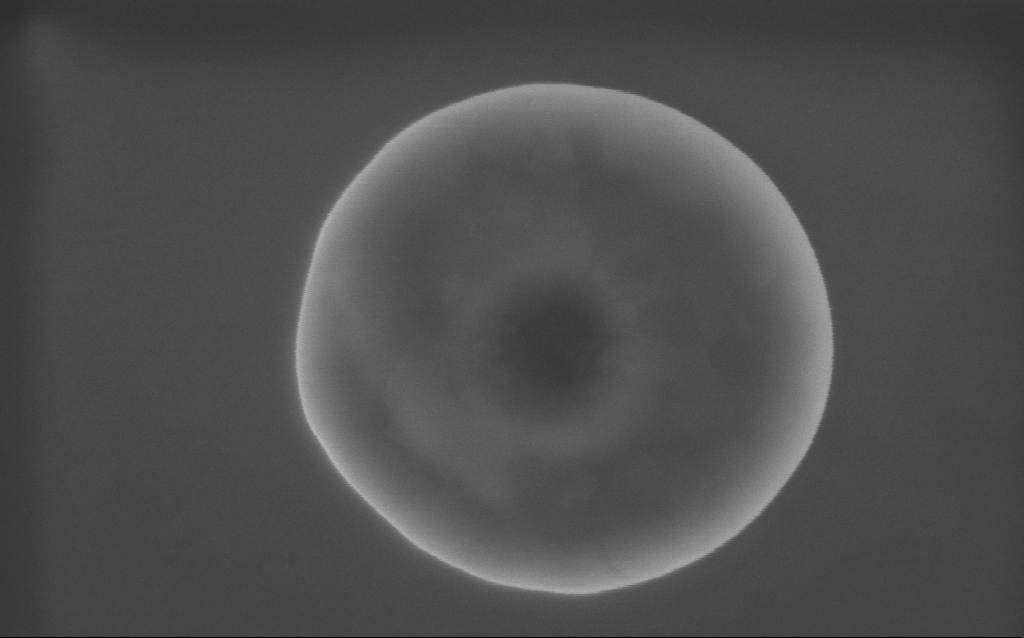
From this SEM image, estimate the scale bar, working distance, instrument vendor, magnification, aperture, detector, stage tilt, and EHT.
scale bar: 100 nm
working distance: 2 mm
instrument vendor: Zeiss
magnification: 157 K X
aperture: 30 µm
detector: InLens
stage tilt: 0°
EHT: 10 kV